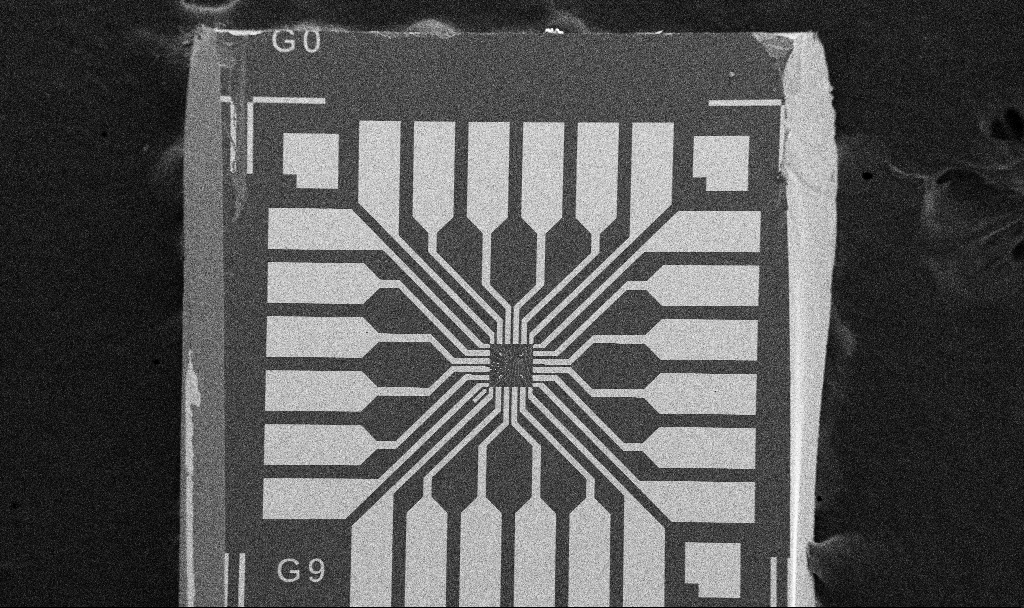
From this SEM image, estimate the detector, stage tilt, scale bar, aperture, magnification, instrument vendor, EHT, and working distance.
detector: SE2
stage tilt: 0°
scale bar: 200000 nm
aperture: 30 µm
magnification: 0.1 K X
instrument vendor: Zeiss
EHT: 5 kV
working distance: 10.7 mm